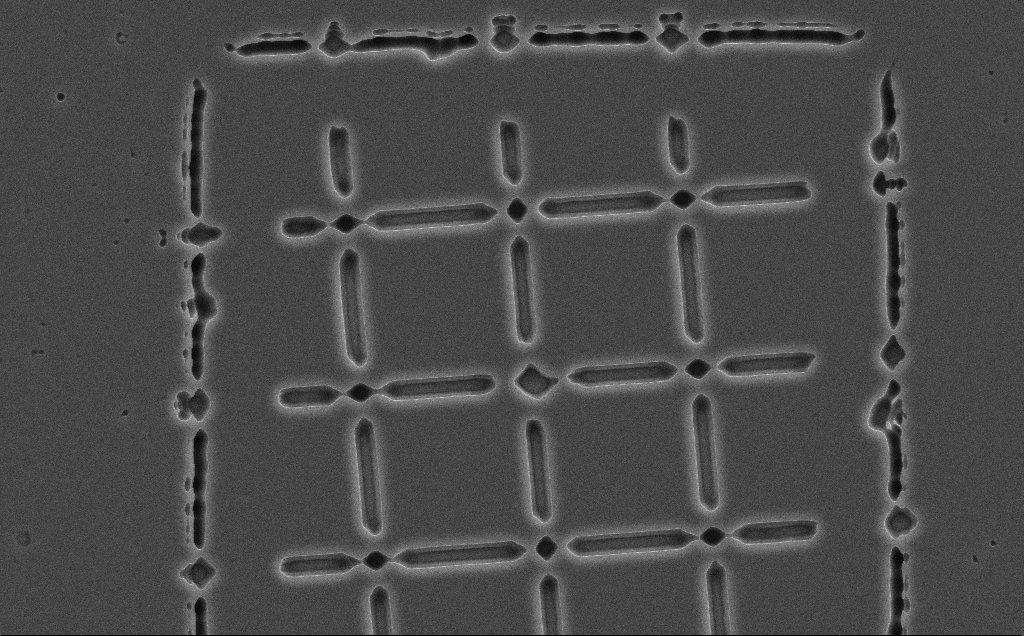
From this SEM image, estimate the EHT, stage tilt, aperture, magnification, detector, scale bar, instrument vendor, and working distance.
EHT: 10 kV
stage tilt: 0°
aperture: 30 µm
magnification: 3.07 K X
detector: SE2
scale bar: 20000 nm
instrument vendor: Zeiss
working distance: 13 mm